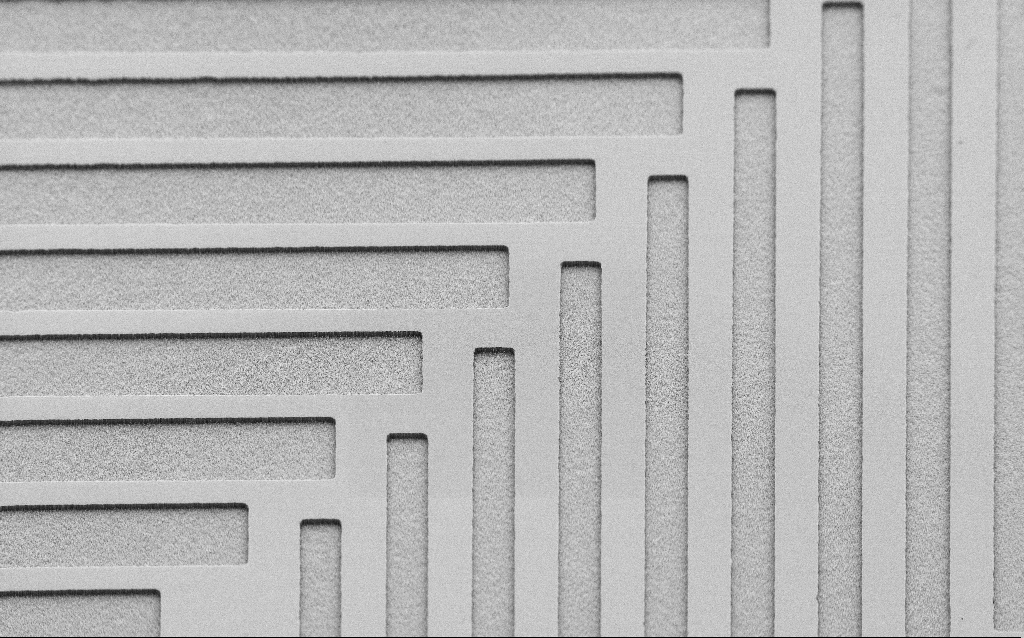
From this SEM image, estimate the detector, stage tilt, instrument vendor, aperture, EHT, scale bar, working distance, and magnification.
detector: SE2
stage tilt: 45°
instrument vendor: Zeiss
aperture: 30 µm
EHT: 2 kV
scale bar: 100000 nm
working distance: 7 mm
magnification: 0.423 K X